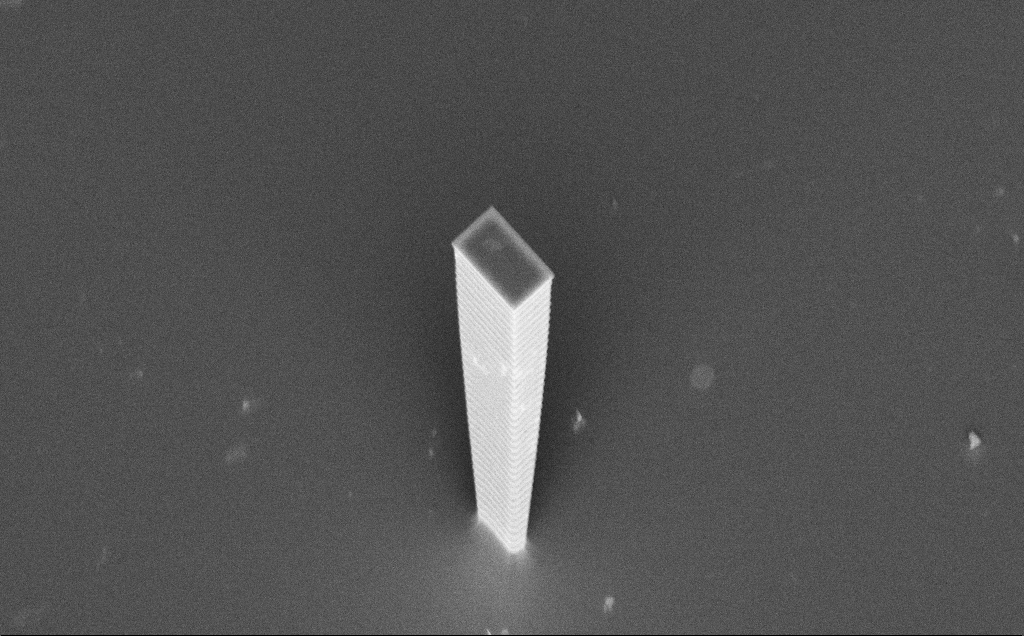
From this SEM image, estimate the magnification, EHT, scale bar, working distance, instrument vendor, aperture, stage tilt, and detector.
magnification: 7.18 K X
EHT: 7.5 kV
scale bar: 10000 nm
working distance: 8 mm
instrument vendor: Zeiss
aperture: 30 µm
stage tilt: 45°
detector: InLens